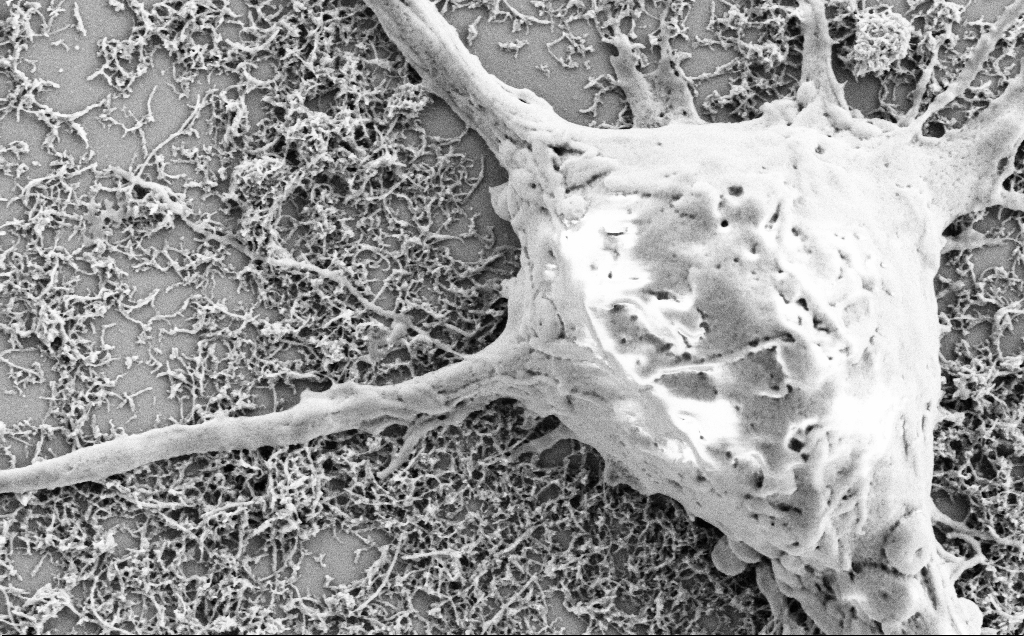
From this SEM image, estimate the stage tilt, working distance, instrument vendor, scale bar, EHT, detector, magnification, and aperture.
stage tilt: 0°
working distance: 7.1 mm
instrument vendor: Zeiss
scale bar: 1000 nm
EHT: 2 kV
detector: SE2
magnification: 25 K X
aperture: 30 µm